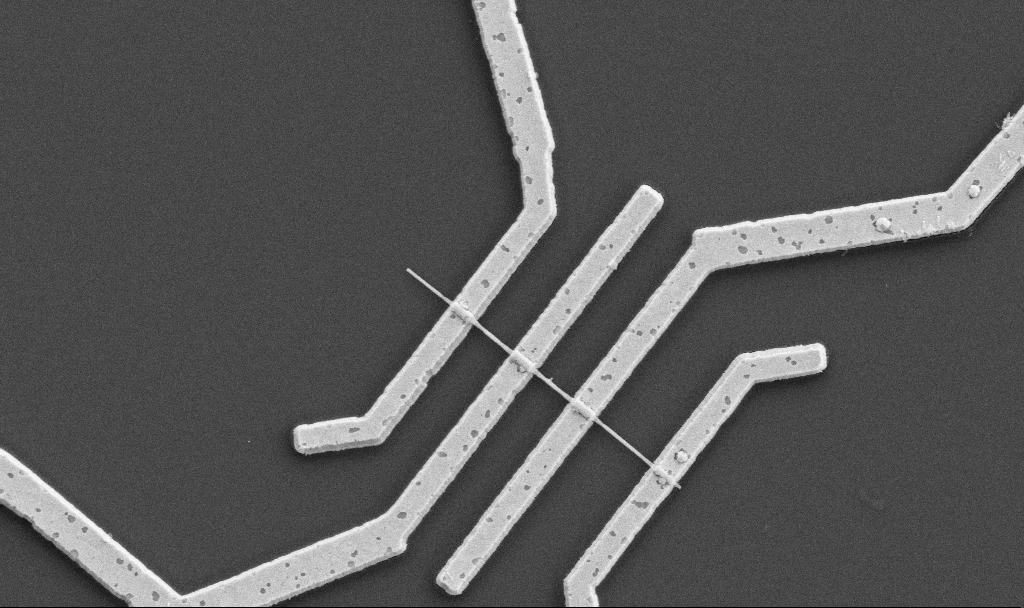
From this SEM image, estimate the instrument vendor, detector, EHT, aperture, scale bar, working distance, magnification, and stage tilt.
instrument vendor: Zeiss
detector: SE2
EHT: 5 kV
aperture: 30 µm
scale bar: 2000 nm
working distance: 10.6 mm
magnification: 20 K X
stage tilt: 0°